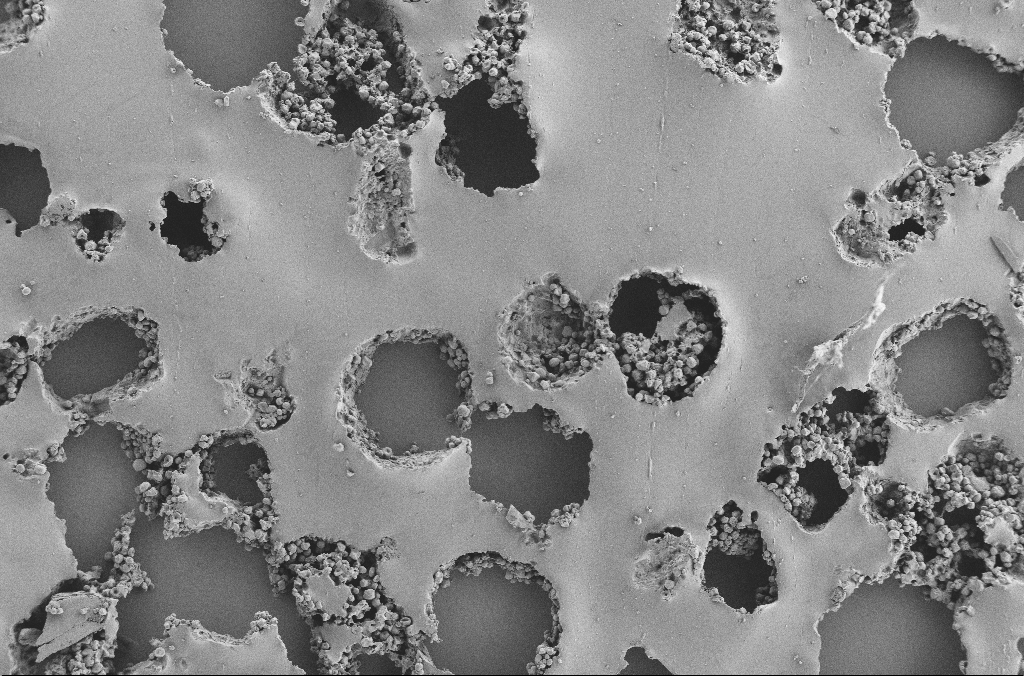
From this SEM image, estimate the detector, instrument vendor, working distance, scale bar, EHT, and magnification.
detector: SE2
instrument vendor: Zeiss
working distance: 3.7 mm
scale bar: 100000 nm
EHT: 2 kV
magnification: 0.25 K X